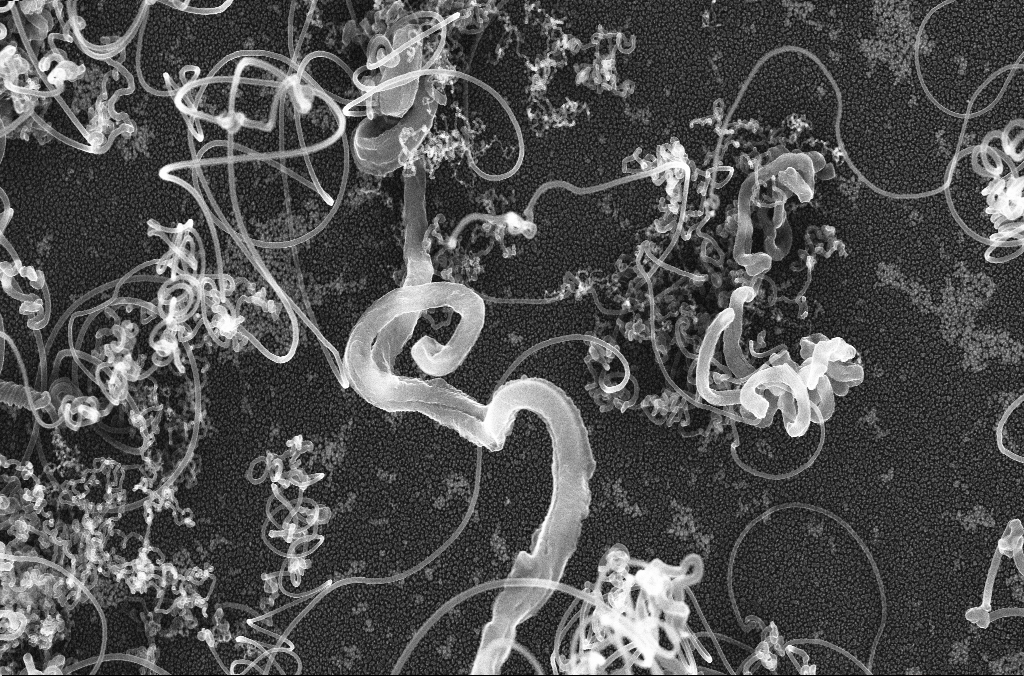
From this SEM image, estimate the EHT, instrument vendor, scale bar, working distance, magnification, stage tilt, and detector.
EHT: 20 kV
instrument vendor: Zeiss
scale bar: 2000 nm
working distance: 4.2 mm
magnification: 16.91 K X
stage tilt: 0°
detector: InLens